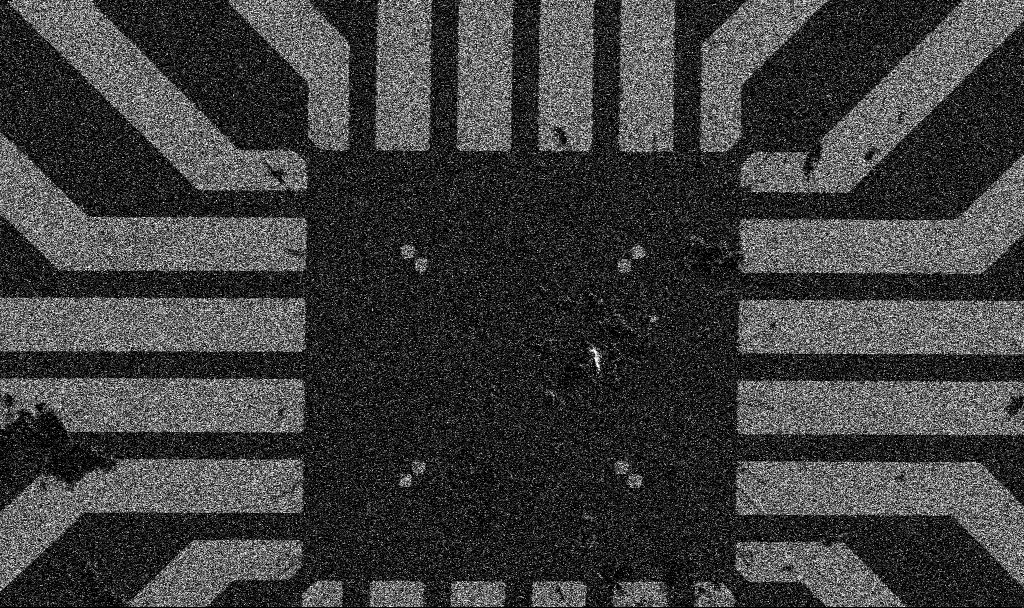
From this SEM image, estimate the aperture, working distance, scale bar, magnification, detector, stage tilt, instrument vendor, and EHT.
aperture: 30 µm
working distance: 8.9 mm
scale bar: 20000 nm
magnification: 1 K X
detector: SE2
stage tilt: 0°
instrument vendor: Zeiss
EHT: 5 kV